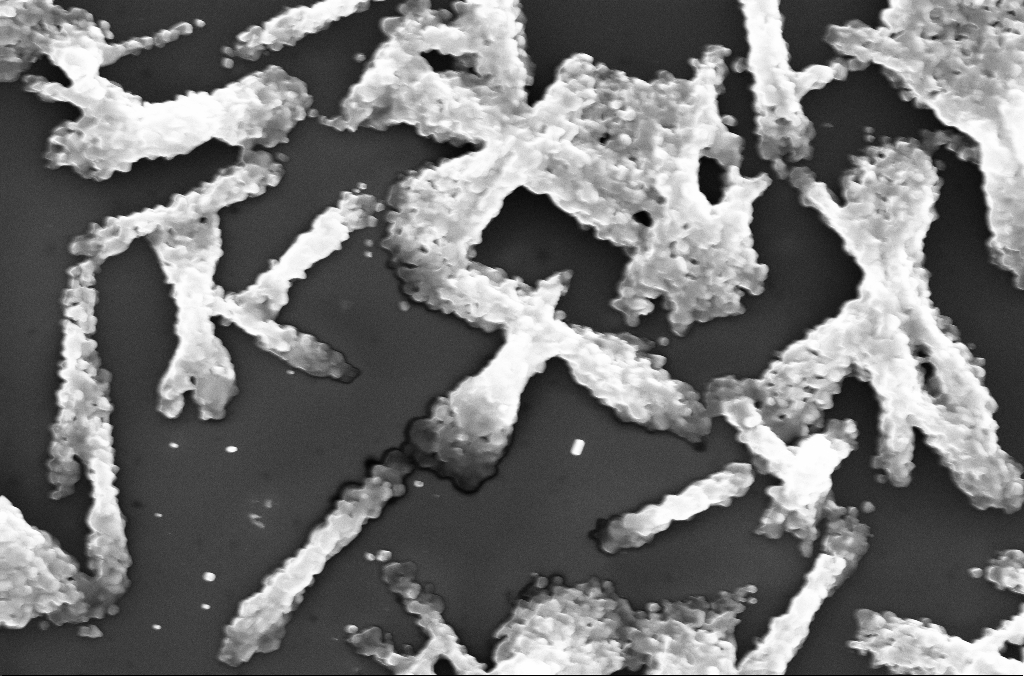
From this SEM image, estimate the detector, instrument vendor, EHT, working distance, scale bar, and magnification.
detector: InLens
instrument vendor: Zeiss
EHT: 20 kV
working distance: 2.8 mm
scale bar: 2000 nm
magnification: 15 K X